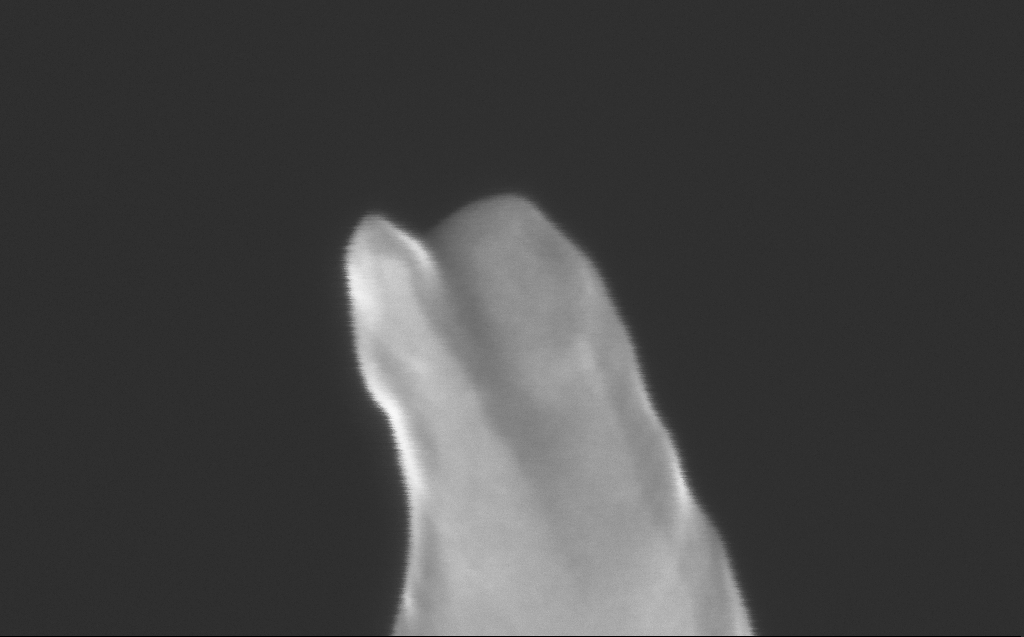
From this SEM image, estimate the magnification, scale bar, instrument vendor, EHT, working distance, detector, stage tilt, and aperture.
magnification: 716.18 K X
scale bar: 100 nm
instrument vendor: Zeiss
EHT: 10 kV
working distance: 4 mm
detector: InLens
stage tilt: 40°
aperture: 30 µm